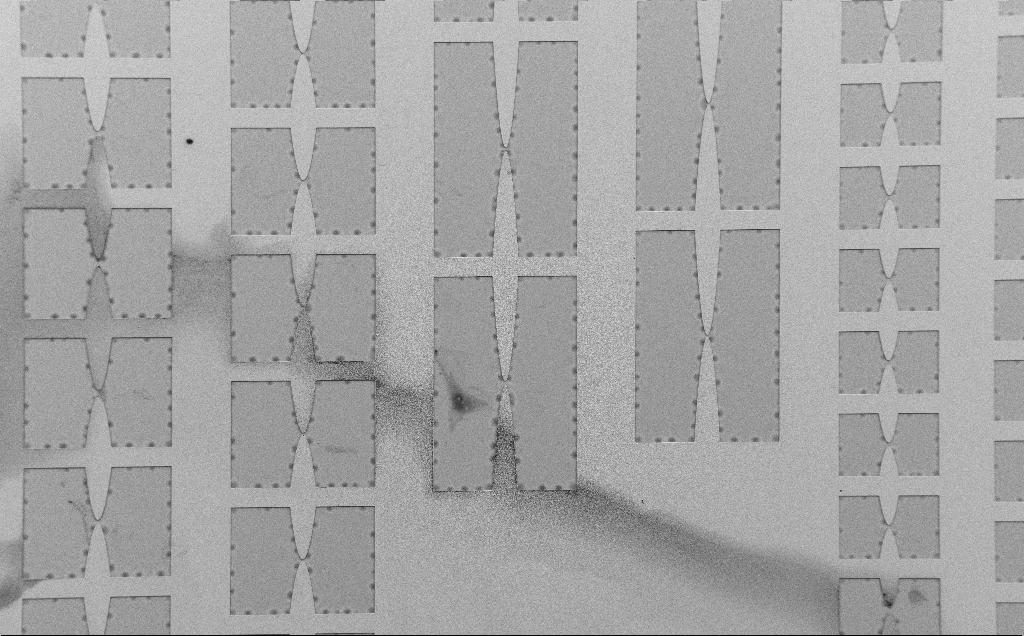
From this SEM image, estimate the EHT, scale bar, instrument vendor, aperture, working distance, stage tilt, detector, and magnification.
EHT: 5 kV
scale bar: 100000 nm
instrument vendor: Zeiss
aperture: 30 µm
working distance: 6 mm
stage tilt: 0°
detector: SE2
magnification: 0.147 K X